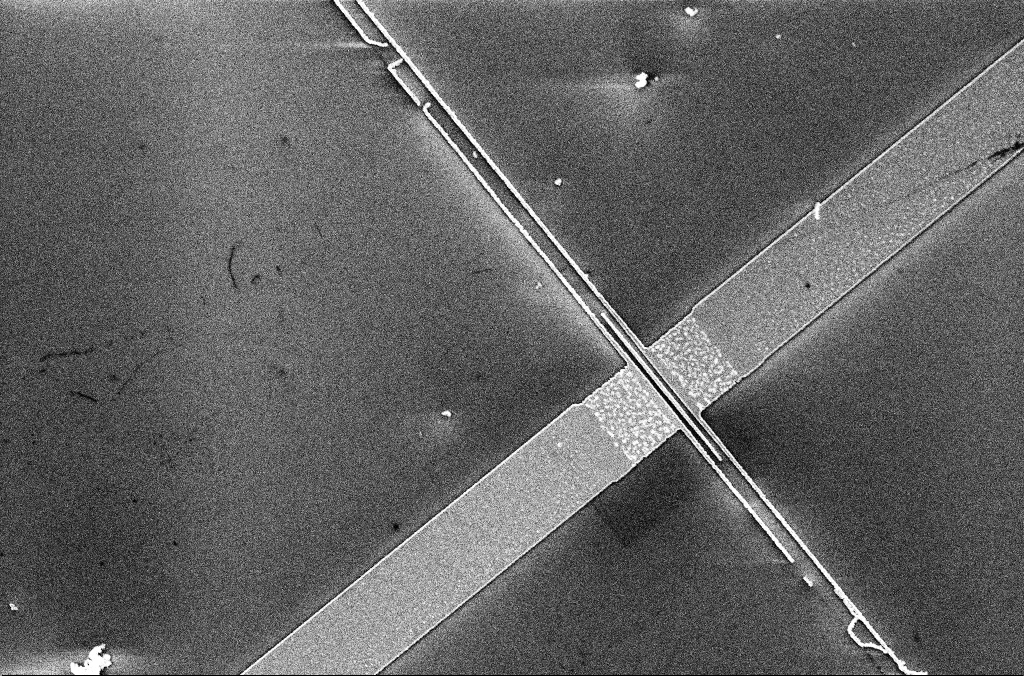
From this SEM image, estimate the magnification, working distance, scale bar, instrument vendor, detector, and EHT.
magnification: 6.25 K X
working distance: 3.3 mm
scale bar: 10000 nm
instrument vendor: Zeiss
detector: InLens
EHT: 5 kV